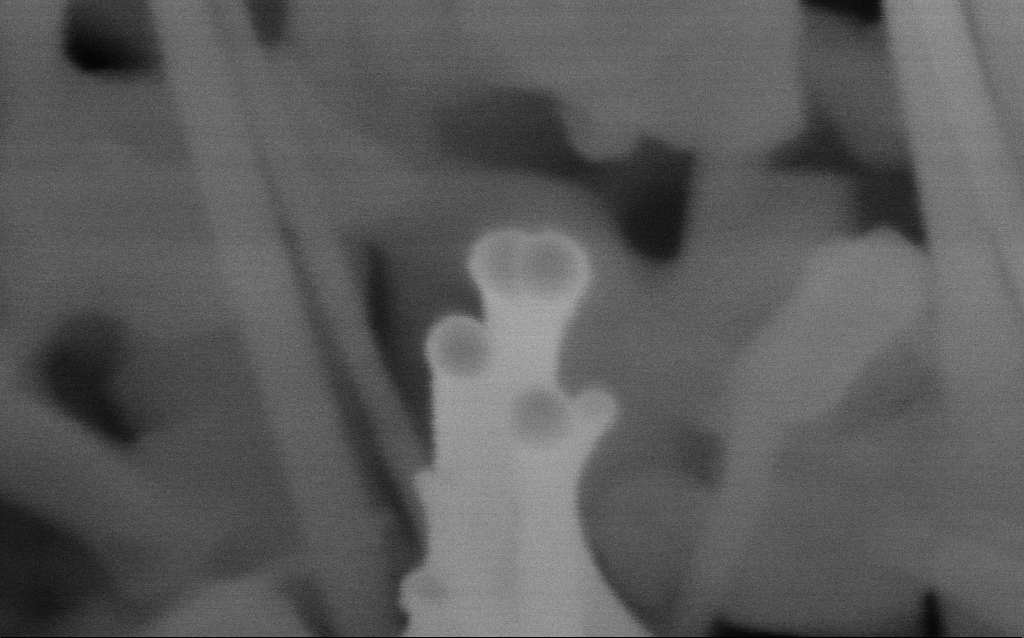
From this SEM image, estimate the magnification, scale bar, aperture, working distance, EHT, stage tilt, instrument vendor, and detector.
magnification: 779.75 K X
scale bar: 20 nm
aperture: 30 µm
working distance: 7.3 mm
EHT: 10 kV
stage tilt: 35°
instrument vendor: Zeiss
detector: InLens